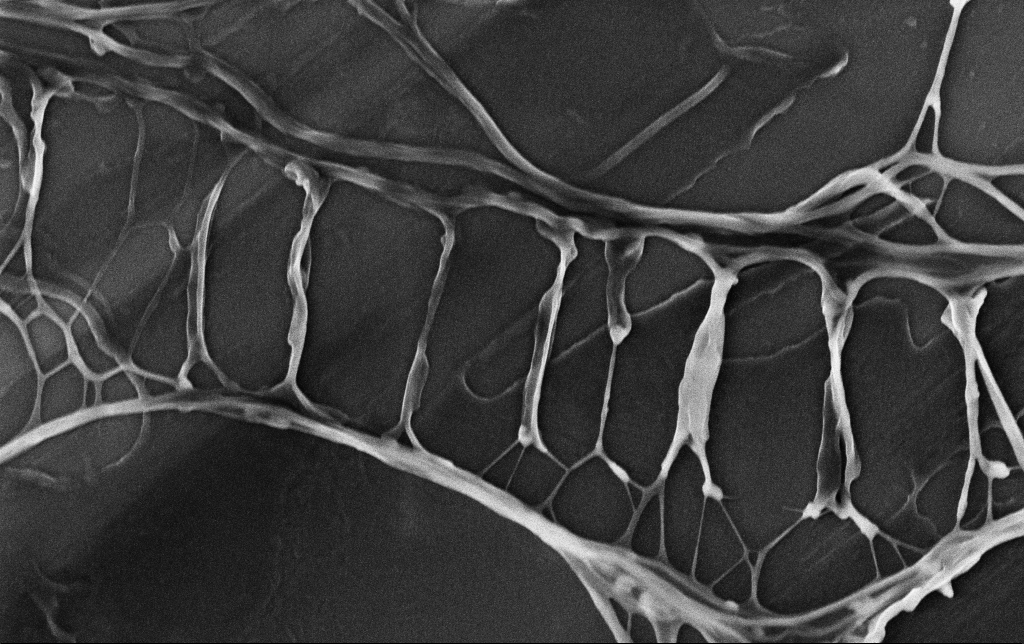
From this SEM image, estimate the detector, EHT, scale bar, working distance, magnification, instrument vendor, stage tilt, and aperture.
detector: InLens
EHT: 3 kV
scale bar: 1000 nm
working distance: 3.5 mm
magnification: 33.61 K X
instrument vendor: Zeiss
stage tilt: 0°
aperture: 30 µm